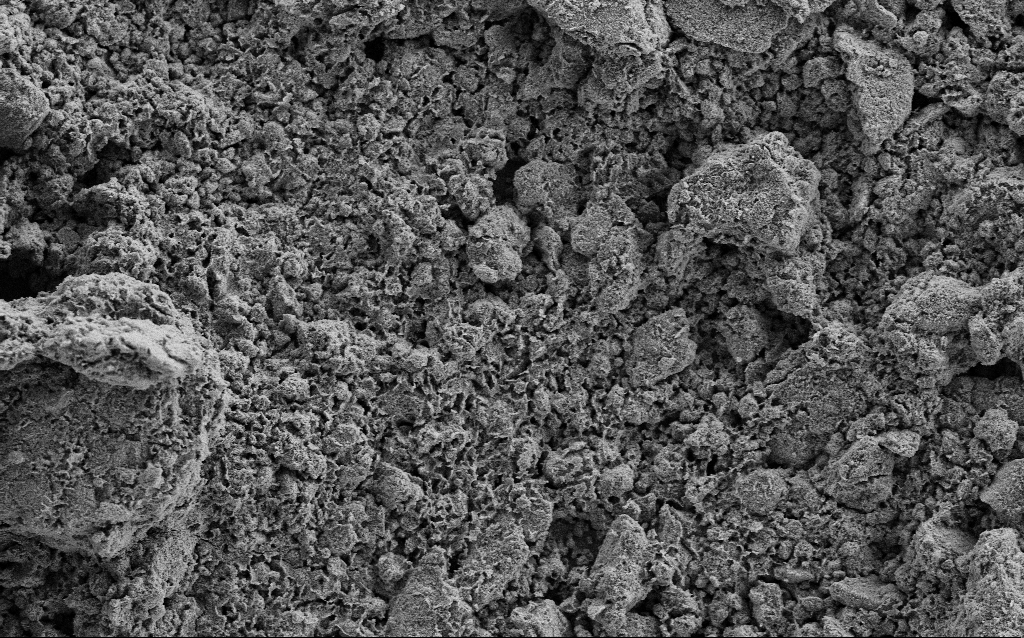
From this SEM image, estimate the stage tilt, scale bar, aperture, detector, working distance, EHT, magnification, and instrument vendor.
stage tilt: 0°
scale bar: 20000 nm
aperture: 30 µm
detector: SE2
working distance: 4.4 mm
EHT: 5 kV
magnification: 1.23 K X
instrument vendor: Zeiss